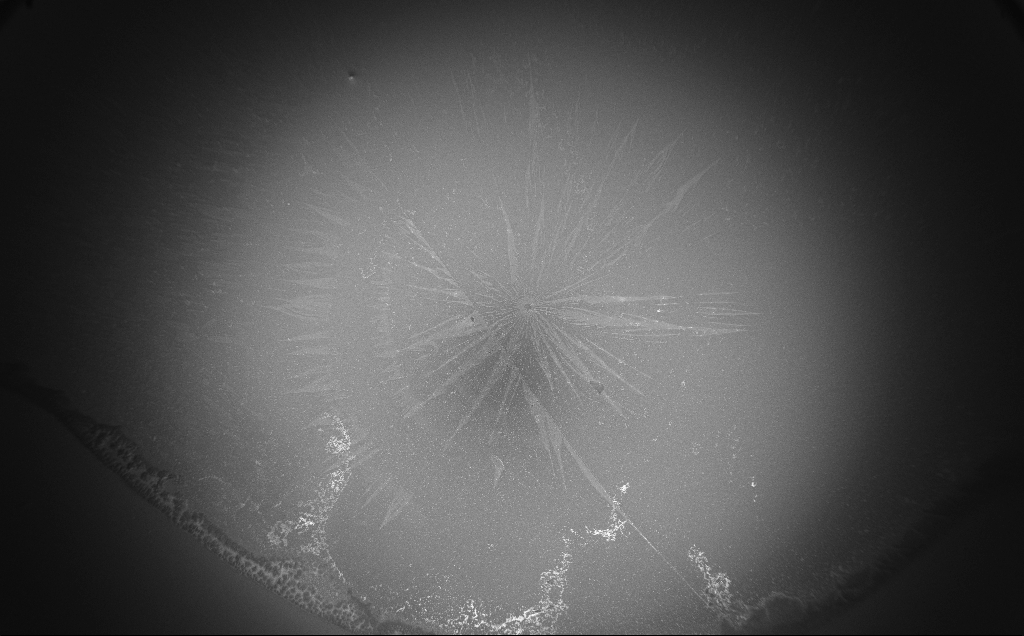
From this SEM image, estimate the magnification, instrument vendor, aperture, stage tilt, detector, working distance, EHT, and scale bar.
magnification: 0.08 K X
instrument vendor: Zeiss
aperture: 30 µm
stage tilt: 0°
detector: InLens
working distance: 5 mm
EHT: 3 kV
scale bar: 200000 nm